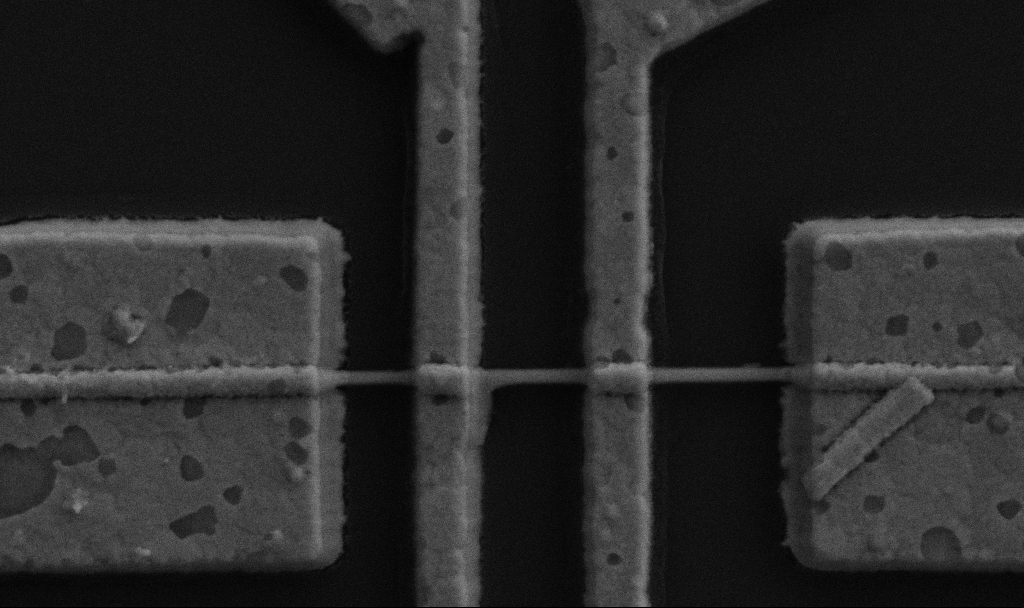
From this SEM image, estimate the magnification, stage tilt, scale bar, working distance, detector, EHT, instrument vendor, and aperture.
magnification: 60 K X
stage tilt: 0°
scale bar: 1000 nm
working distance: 9.7 mm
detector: SE2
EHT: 5 kV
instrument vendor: Zeiss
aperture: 30 µm